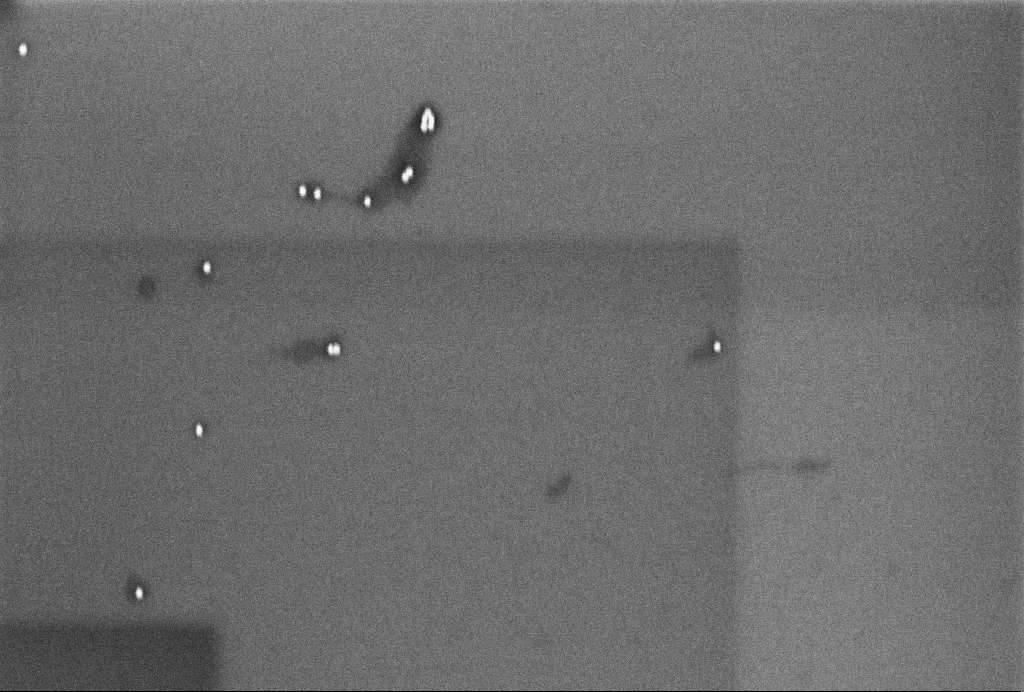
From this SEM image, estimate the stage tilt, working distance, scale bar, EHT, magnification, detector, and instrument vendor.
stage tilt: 0°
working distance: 3.3 mm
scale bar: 200 nm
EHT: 2 kV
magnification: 100.38 K X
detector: InLens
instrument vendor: Zeiss